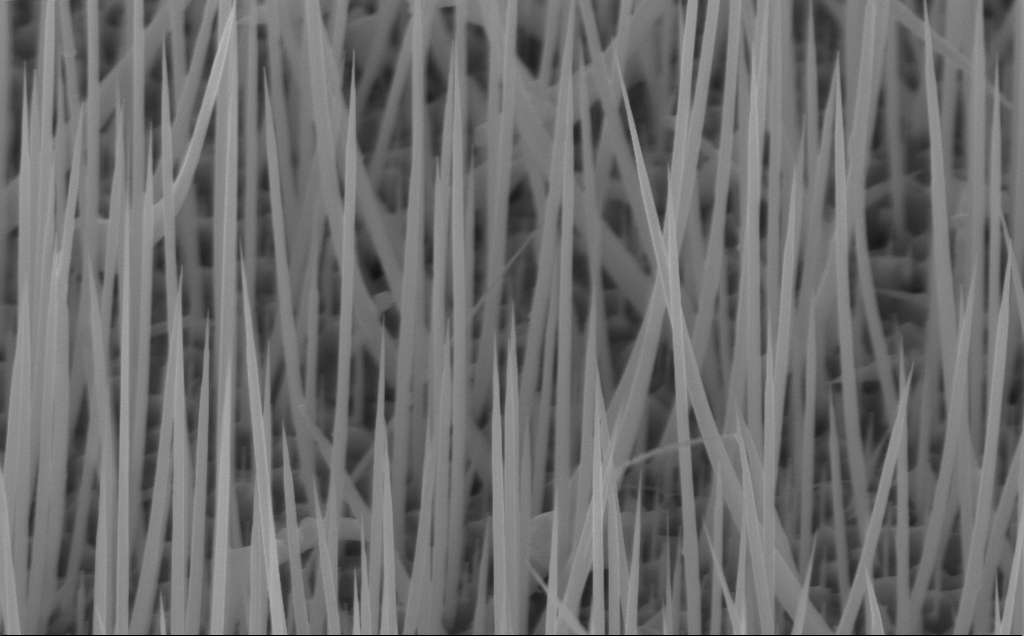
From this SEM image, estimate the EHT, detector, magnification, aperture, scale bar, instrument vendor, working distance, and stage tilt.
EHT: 10 kV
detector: InLens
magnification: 40 K X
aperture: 30 µm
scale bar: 1000 nm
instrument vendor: Zeiss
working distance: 7 mm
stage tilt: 45°